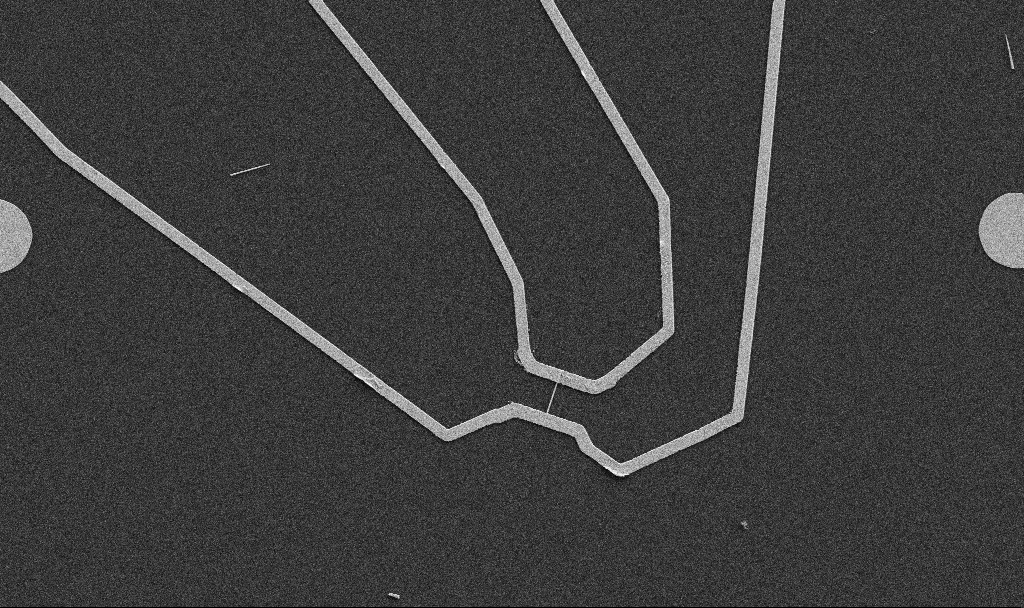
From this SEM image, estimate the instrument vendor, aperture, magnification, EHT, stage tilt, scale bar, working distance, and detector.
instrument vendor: Zeiss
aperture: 30 µm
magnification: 5 K X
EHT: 5 kV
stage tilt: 0°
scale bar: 10000 nm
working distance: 10.7 mm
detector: SE2